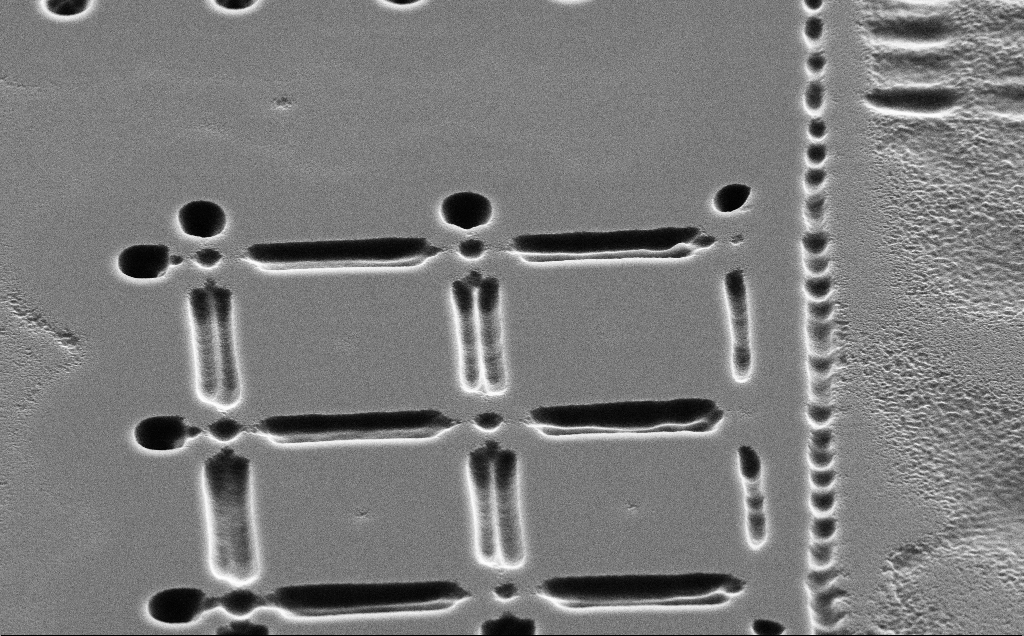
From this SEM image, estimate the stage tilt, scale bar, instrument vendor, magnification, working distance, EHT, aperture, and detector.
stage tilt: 45°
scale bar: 2000 nm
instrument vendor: Zeiss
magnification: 9.47 K X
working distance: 10 mm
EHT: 5 kV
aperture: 30 µm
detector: SE2